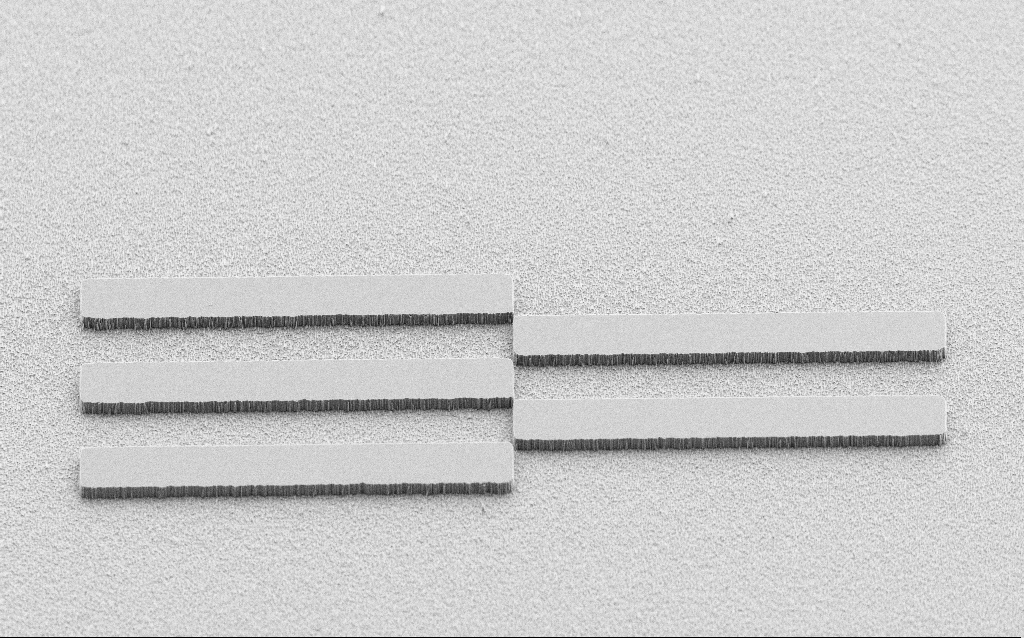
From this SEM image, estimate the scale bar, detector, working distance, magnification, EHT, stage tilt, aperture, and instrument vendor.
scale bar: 20000 nm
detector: SE2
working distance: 7 mm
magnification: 0.8 K X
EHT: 5 kV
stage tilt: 45°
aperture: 30 µm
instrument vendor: Zeiss